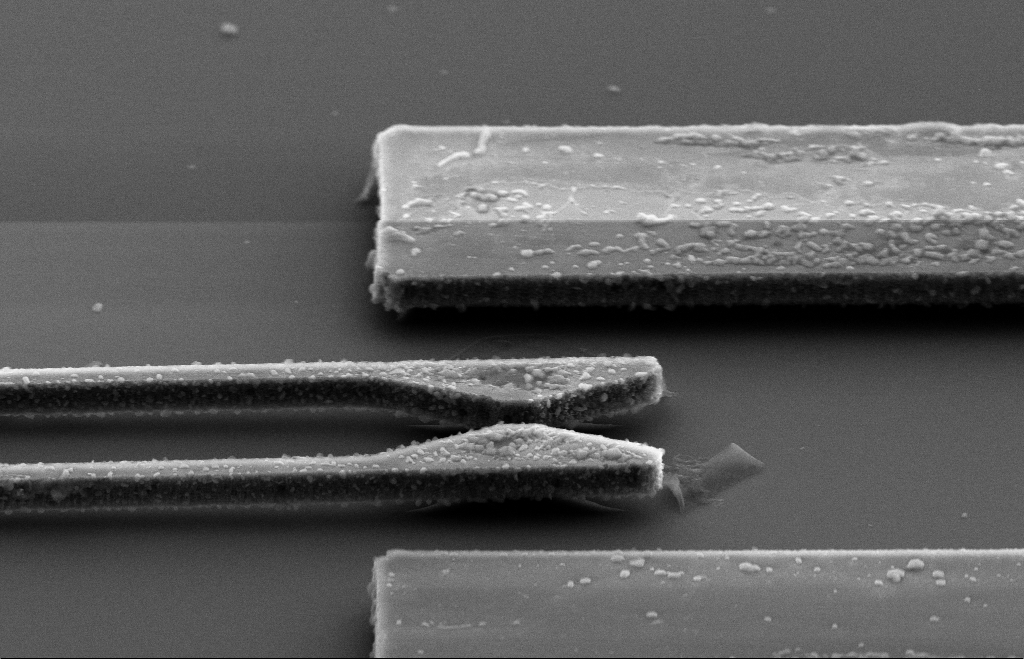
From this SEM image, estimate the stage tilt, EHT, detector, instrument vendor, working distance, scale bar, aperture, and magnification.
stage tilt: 57.1°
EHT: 10 kV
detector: SE2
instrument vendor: Zeiss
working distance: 9 mm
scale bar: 2000 nm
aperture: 30 µm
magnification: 5.45 K X